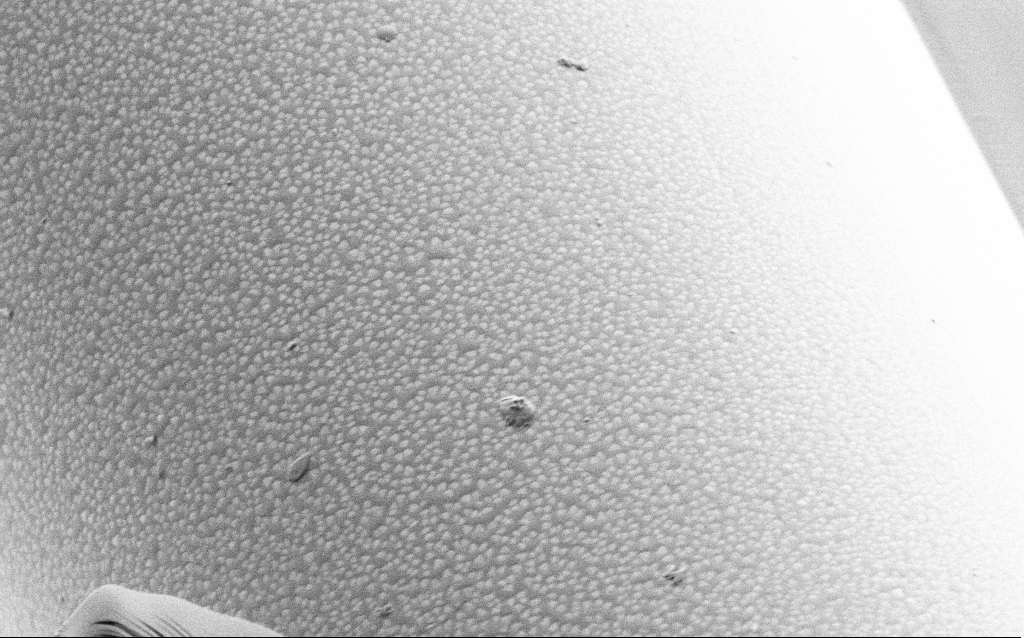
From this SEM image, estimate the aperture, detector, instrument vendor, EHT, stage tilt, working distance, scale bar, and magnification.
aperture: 30 µm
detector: SE2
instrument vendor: Zeiss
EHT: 1 kV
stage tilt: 45°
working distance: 7.7 mm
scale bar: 2000 nm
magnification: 25 K X